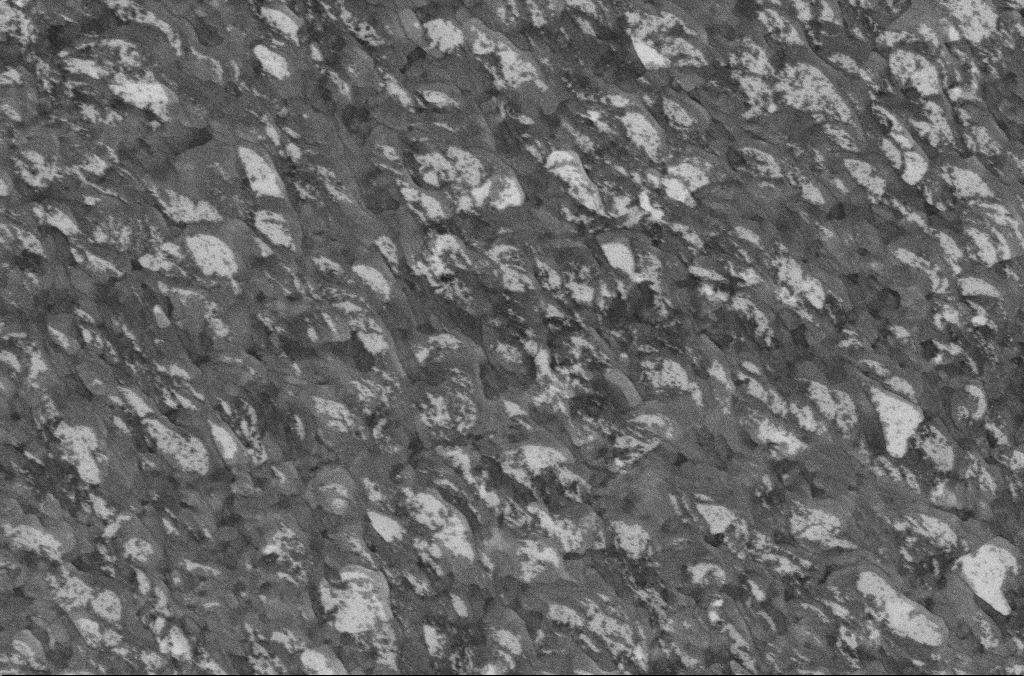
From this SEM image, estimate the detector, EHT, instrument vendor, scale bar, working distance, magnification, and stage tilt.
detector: InLens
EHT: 5 kV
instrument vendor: Zeiss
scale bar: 1000 nm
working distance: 6.7 mm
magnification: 20 K X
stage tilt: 0°